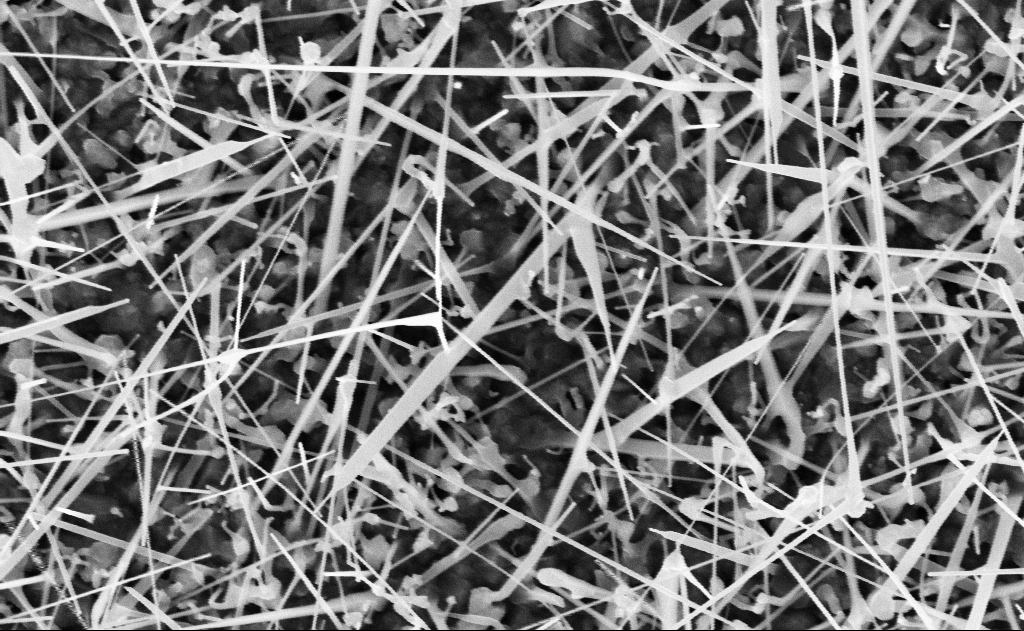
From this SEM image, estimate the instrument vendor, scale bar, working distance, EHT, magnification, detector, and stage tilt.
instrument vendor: Zeiss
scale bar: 1000 nm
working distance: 20 mm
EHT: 10 kV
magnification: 40 K X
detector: InLens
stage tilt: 0°